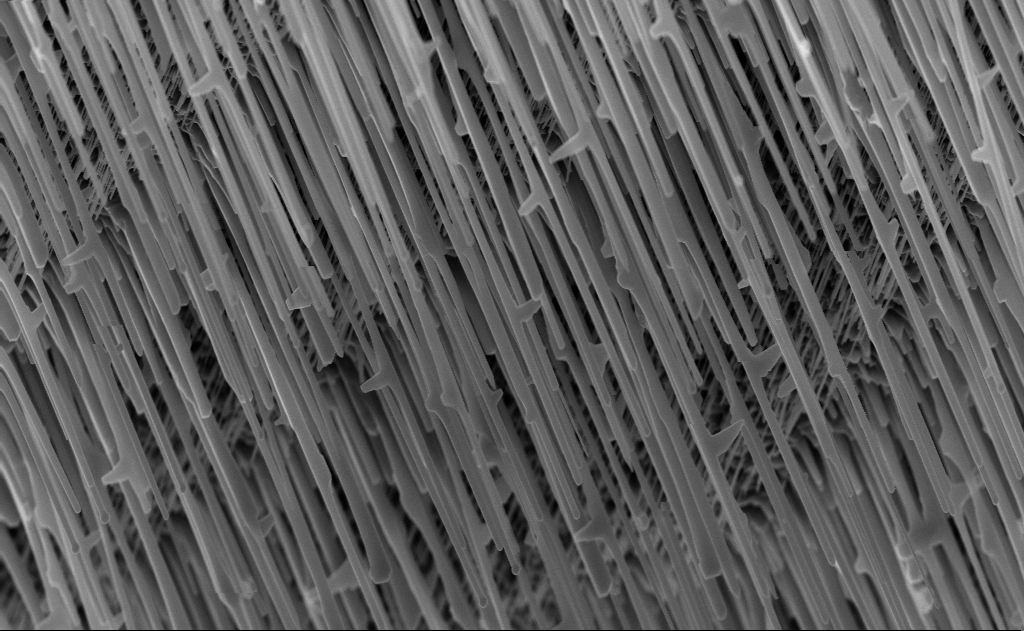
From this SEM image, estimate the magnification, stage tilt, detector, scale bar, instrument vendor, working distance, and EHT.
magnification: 20 K X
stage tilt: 0°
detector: InLens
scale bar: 2000 nm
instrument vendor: Zeiss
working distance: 7 mm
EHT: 10 kV